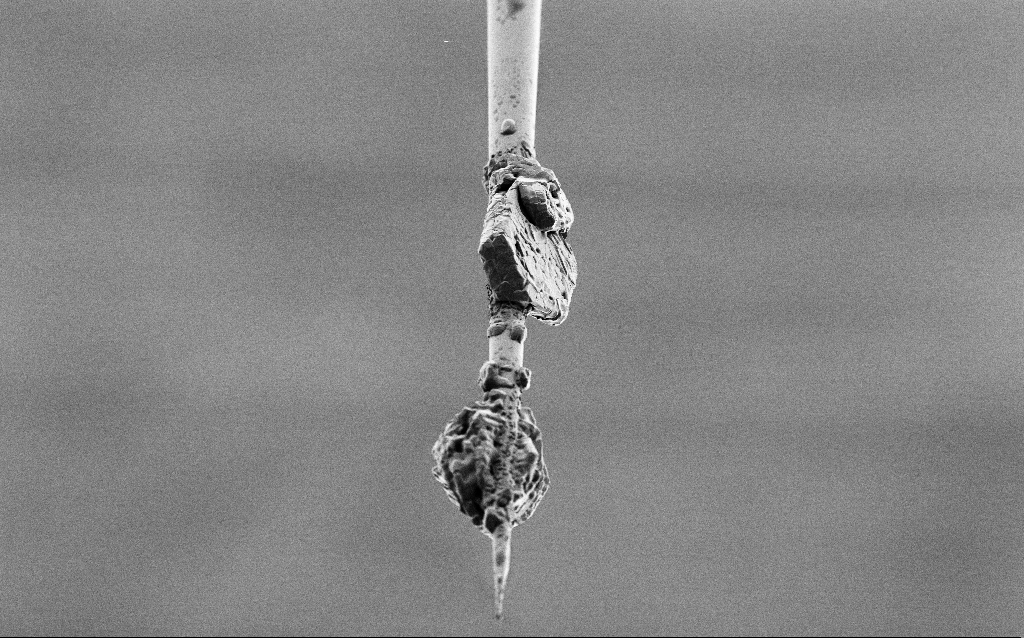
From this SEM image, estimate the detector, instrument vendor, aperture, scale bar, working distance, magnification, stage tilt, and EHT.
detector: SE2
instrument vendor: Zeiss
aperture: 30 µm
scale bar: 100000 nm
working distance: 6.6 mm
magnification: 0.5 K X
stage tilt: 45°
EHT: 1 kV